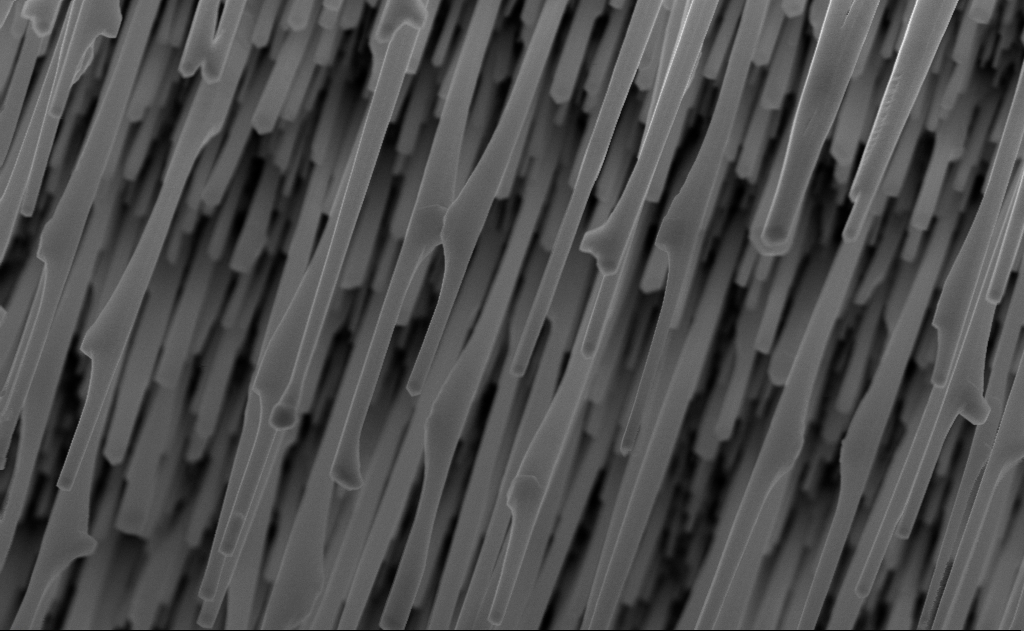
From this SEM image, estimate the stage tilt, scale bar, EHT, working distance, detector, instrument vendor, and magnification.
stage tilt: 0°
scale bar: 1000 nm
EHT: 10 kV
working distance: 9 mm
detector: InLens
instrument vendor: Zeiss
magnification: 40 K X